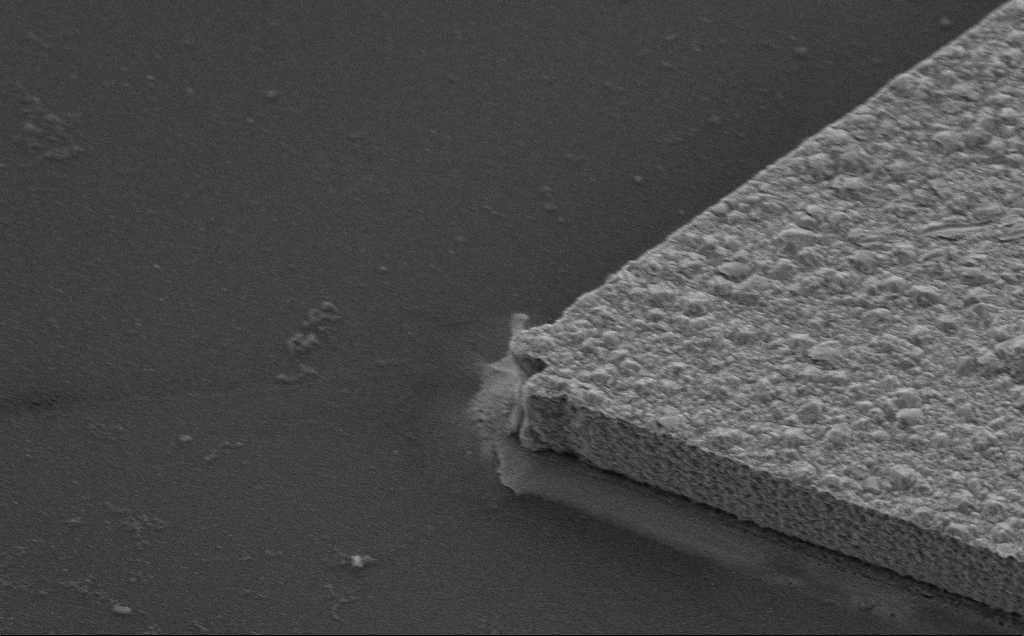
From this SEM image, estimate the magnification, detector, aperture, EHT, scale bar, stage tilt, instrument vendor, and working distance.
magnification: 8.95 K X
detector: SE2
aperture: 30 µm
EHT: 10 kV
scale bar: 2000 nm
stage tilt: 35°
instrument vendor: Zeiss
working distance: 8 mm